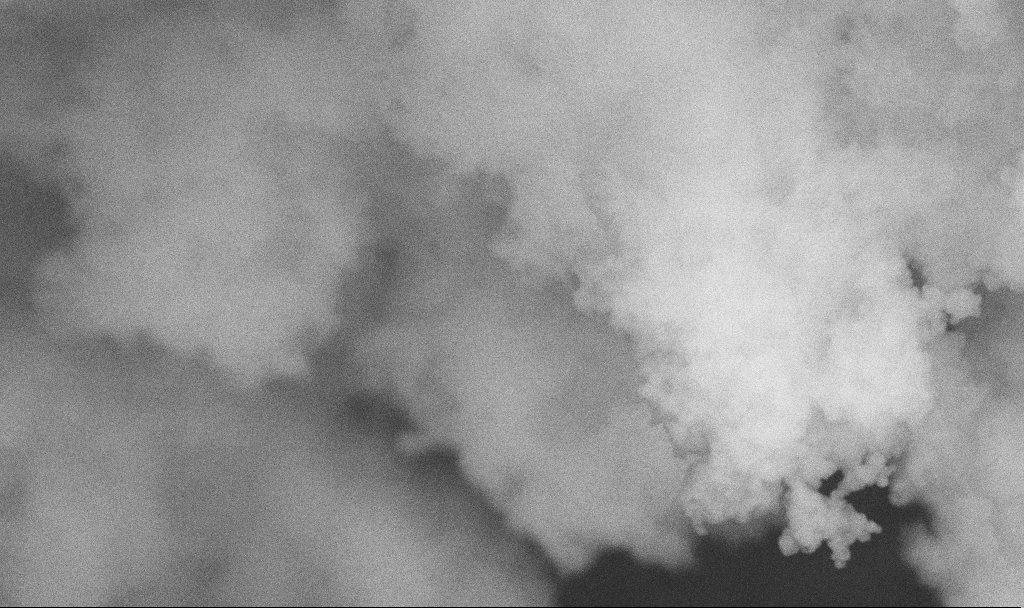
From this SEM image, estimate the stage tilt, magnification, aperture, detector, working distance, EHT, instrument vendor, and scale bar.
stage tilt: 0°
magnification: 130.33 K X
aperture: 30 µm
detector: SE2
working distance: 2.7 mm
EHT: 10 kV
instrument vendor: Zeiss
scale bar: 200 nm